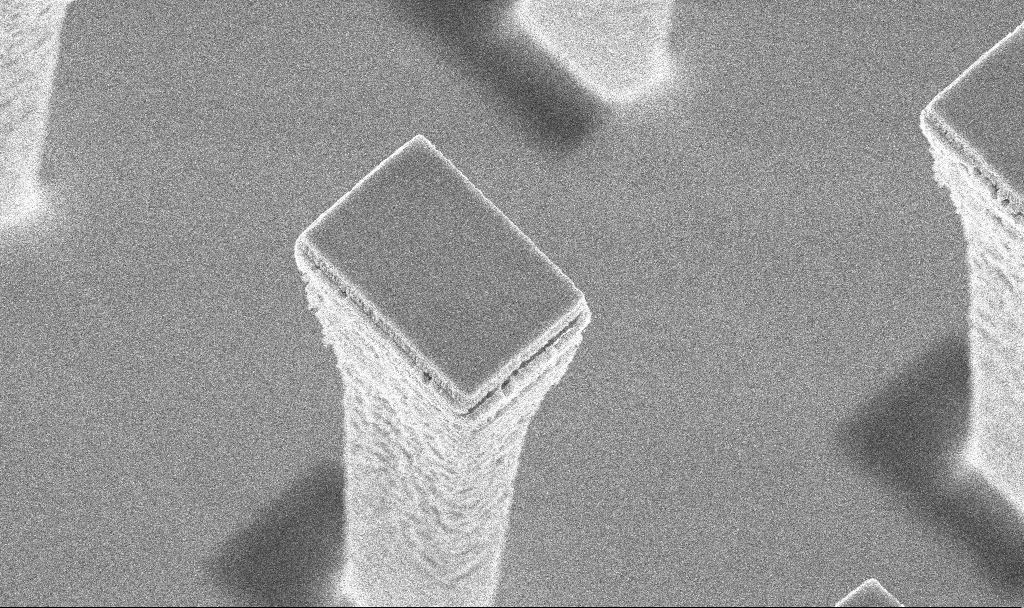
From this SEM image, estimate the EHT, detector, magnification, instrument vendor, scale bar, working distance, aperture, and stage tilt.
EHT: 5 kV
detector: InLens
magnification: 20.09 K X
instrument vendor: Zeiss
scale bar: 2000 nm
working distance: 4.2 mm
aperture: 30 µm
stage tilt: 20°